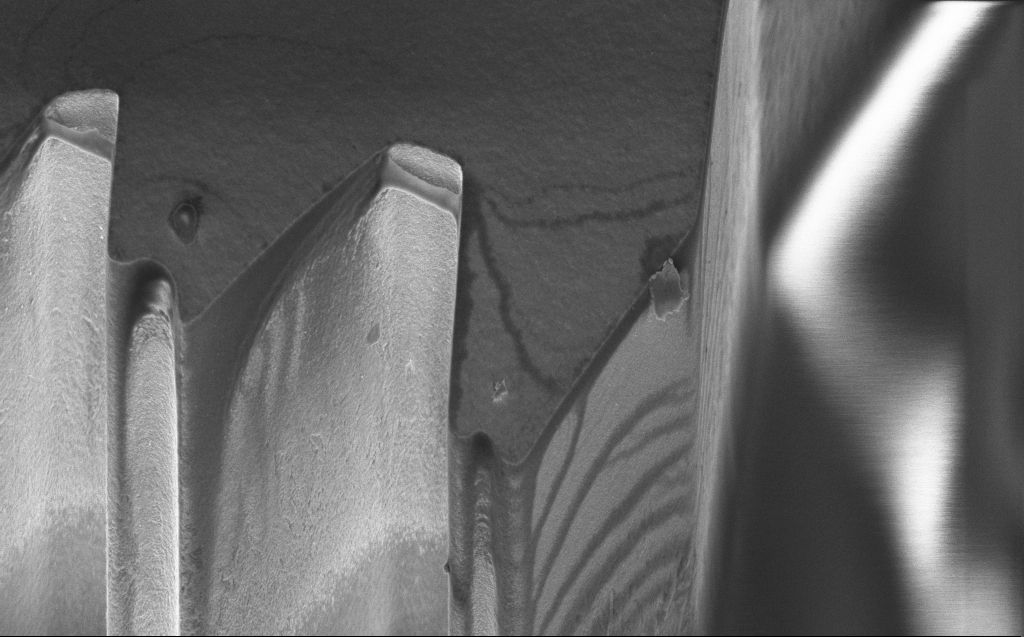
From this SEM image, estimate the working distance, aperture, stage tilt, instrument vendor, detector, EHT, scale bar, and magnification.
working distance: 8 mm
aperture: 30 µm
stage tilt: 45.1°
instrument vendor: Zeiss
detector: InLens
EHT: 3 kV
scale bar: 2000 nm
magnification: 11.17 K X